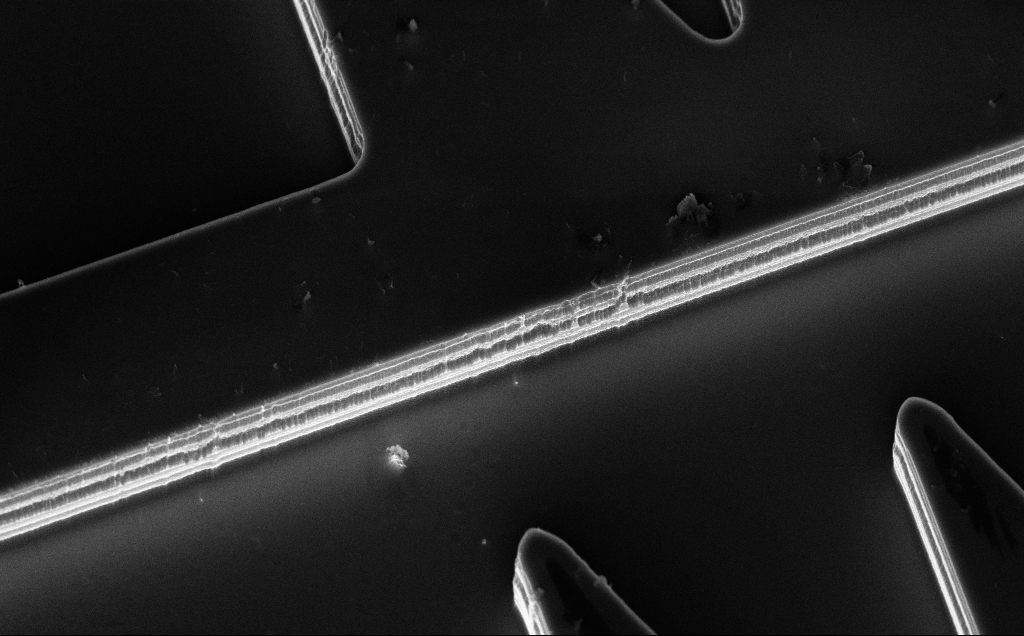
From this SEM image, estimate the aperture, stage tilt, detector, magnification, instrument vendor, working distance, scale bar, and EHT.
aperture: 30 µm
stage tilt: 50°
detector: InLens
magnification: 4.64 K X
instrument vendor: Zeiss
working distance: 11 mm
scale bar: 10000 nm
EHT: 10 kV